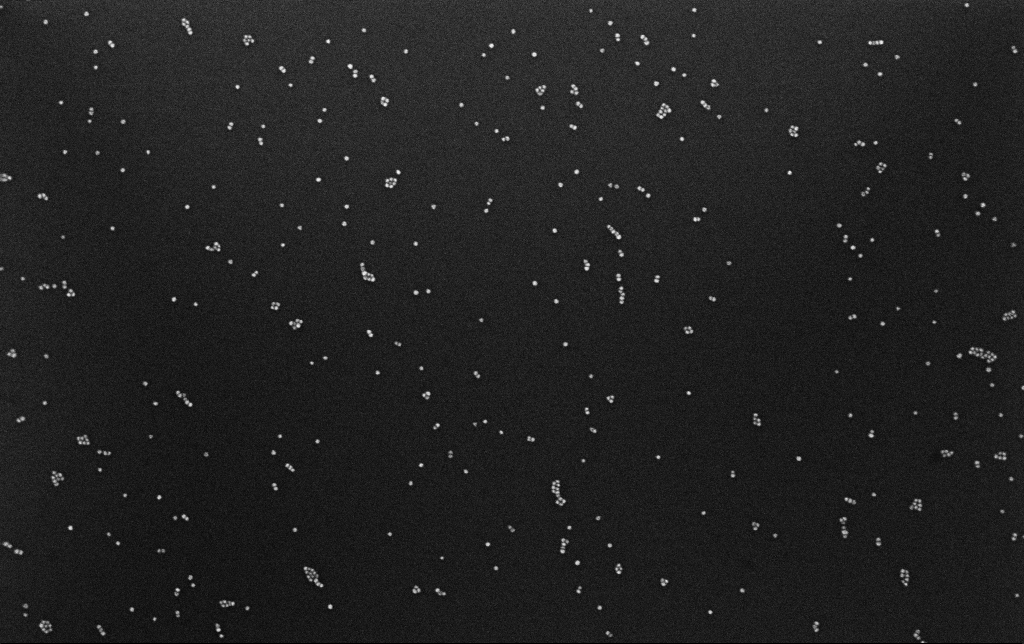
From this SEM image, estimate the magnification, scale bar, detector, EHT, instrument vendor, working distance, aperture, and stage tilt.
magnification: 100 K X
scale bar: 200 nm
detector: InLens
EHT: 10 kV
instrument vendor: Zeiss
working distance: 3.1 mm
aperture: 30 µm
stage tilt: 0°